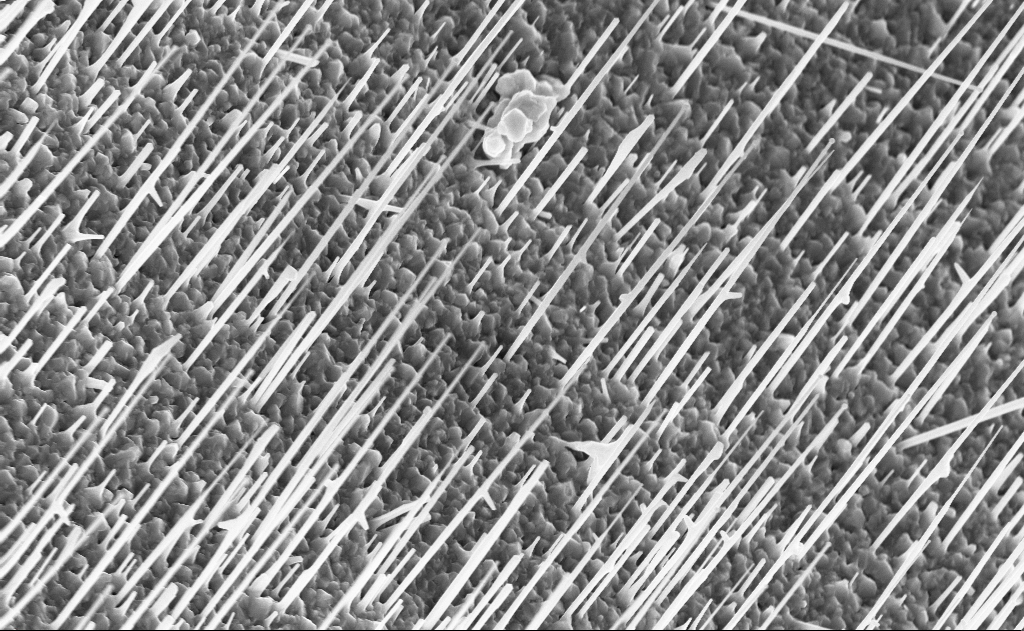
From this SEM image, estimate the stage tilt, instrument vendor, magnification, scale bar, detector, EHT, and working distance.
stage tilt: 0°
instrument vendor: Zeiss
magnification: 20 K X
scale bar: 2000 nm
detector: InLens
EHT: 10 kV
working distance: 10 mm